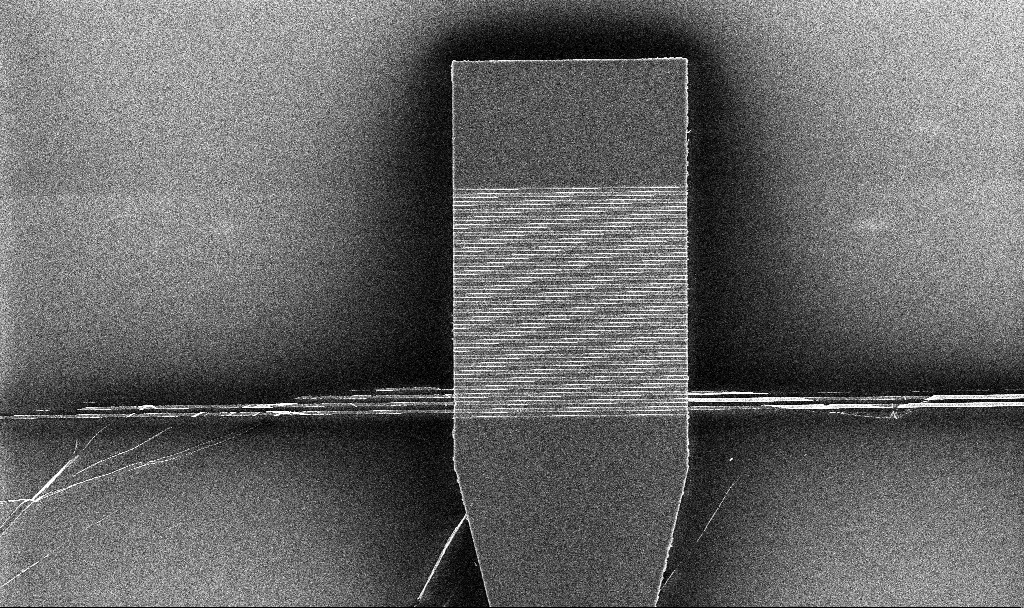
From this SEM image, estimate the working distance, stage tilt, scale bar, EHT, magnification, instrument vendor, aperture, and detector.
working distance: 5.2 mm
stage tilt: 0°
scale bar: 10000 nm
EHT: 5 kV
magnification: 4.34 K X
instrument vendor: Zeiss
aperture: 30 µm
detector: InLens